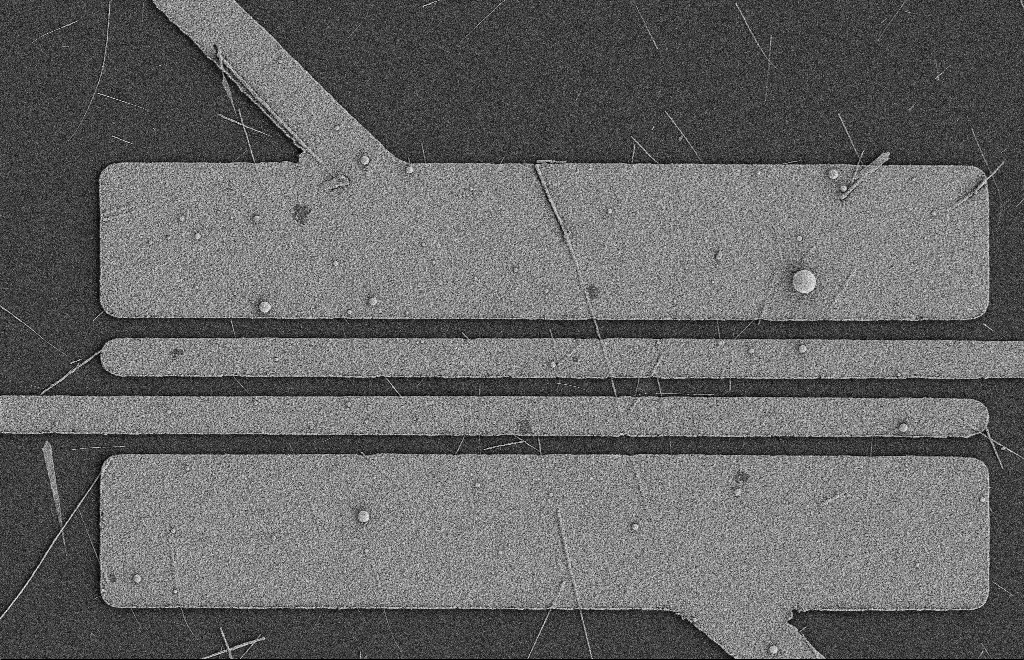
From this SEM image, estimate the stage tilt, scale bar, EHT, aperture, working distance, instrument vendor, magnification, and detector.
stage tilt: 0°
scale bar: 2000 nm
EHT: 2 kV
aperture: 20 µm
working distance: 9 mm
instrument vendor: Zeiss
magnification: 5.35 K X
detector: SE2